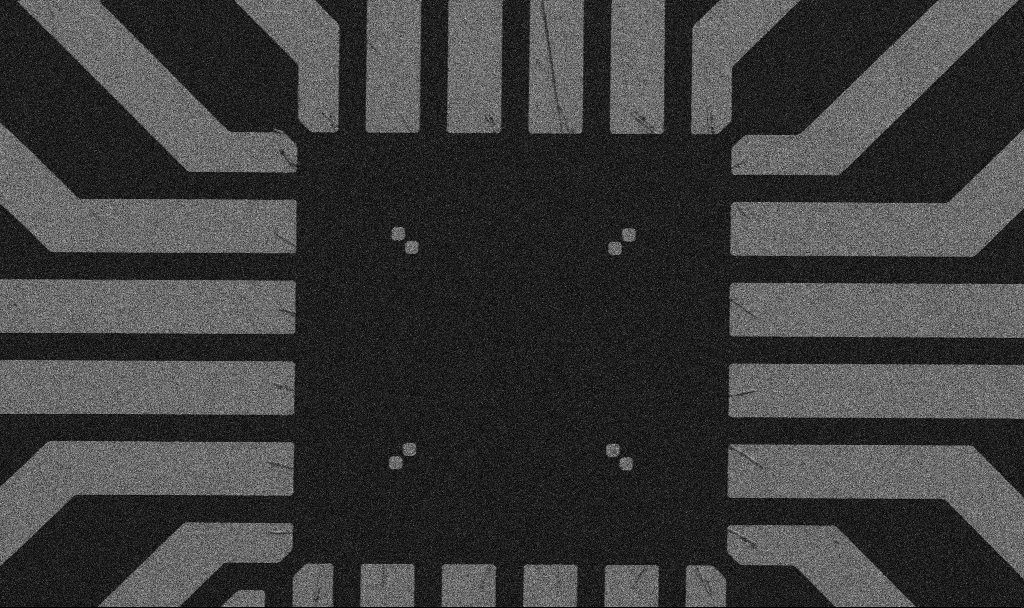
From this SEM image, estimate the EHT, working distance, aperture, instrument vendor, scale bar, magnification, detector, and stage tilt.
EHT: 5 kV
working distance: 9 mm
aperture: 30 µm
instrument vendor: Zeiss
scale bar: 20000 nm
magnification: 1 K X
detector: SE2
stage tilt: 0°